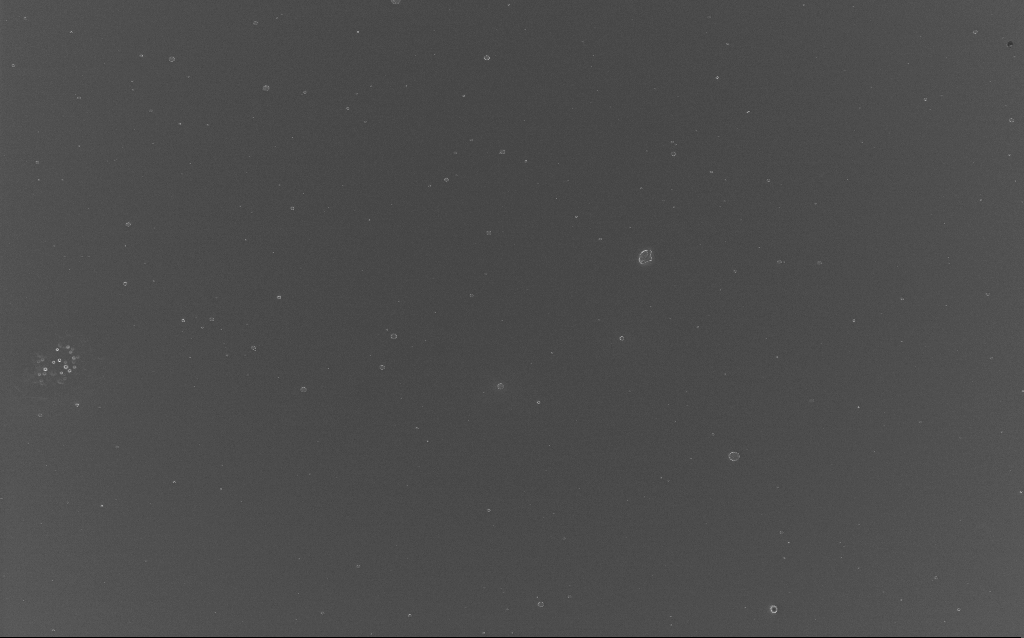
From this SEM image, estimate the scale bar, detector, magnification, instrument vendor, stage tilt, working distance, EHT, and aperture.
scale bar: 20000 nm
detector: InLens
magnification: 1.28 K X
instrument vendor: Zeiss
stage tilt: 0°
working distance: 2 mm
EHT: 5 kV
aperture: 30 µm